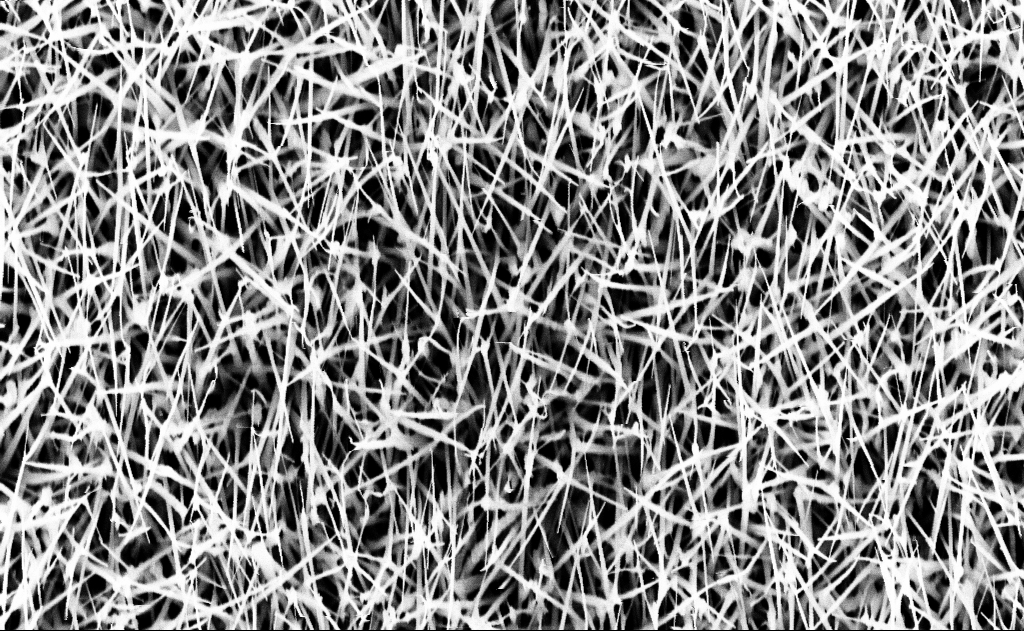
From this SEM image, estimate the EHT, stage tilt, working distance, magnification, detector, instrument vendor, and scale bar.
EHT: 10 kV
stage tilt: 0°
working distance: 16 mm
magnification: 20 K X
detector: InLens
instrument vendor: Zeiss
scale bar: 1000 nm